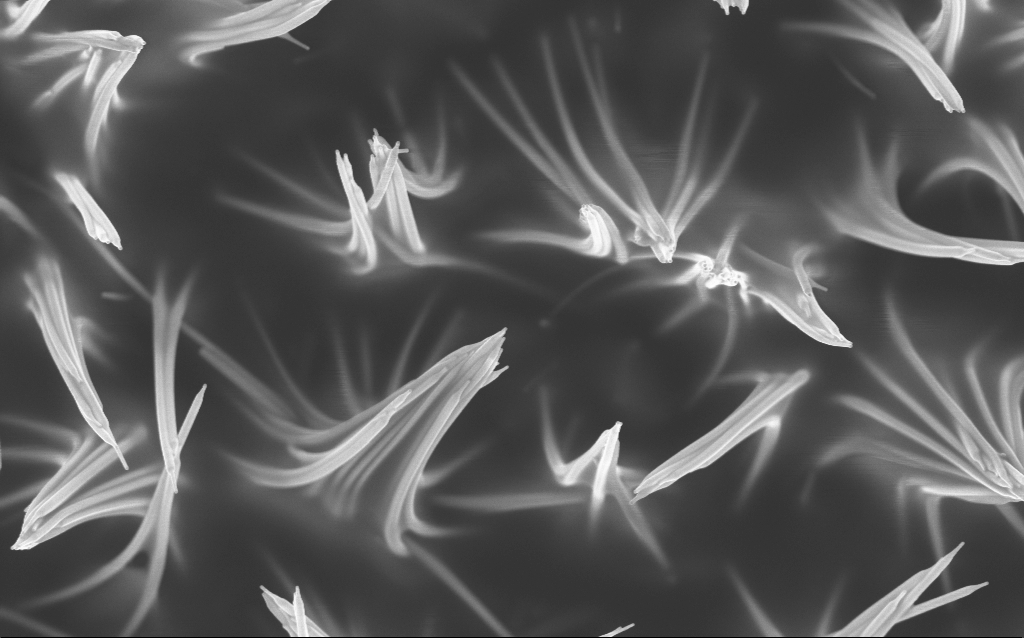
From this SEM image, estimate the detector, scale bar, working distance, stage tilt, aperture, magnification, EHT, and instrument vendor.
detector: InLens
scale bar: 1000 nm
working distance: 3.1 mm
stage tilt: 0°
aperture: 30 µm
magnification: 42.49 K X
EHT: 5 kV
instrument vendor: Zeiss